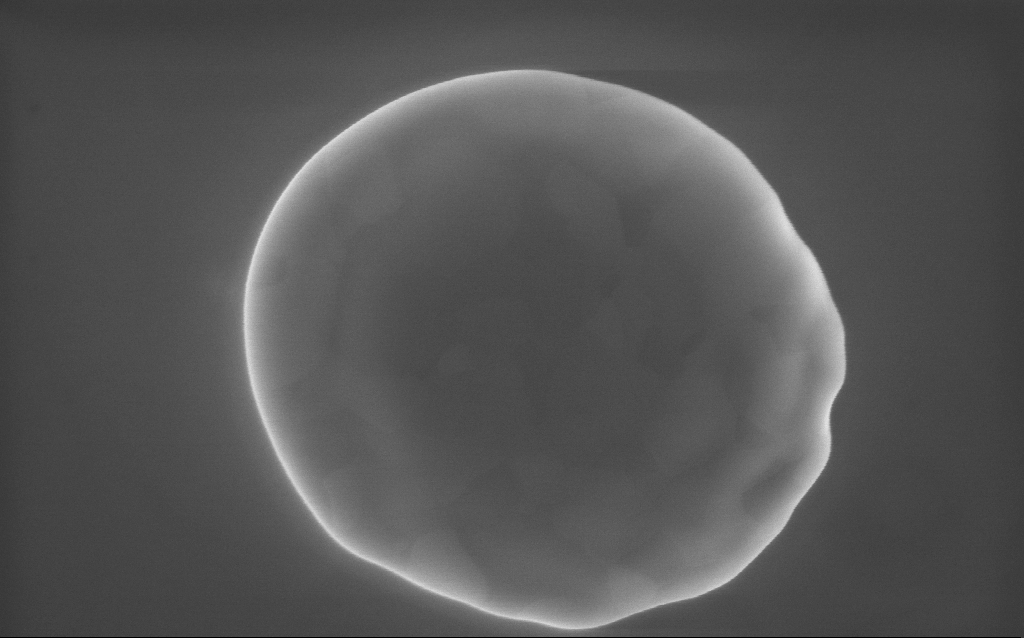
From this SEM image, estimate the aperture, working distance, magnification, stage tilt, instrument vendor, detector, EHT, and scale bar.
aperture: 30 µm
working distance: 2 mm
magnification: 90 K X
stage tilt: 0°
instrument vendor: Zeiss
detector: InLens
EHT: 10 kV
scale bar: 200 nm